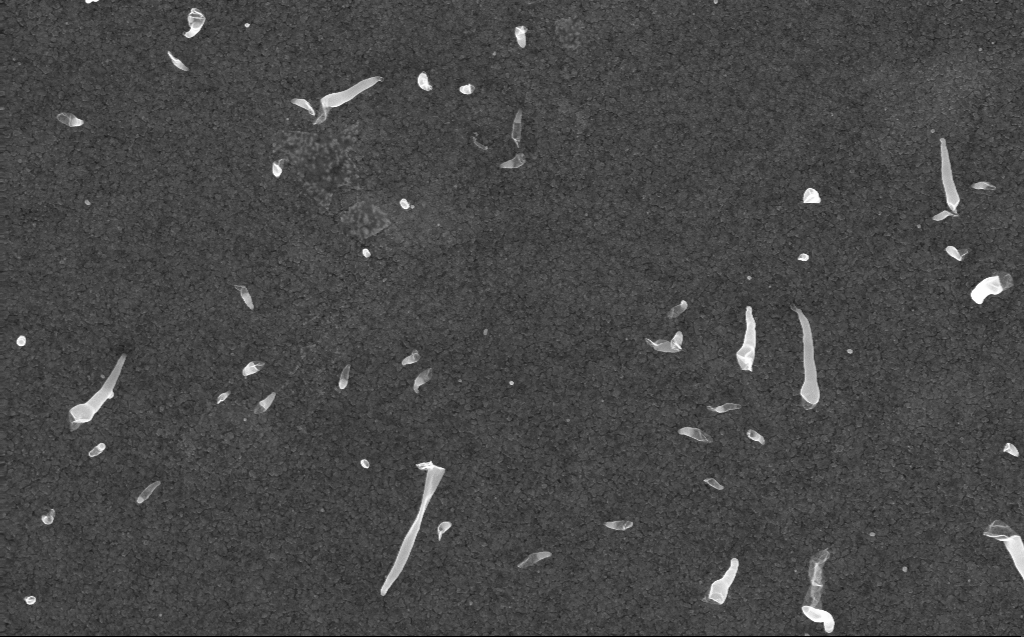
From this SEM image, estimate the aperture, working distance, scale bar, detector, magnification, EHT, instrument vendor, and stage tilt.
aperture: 30 µm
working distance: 3 mm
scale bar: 1000 nm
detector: InLens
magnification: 50 K X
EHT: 10 kV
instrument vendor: Zeiss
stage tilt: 0°